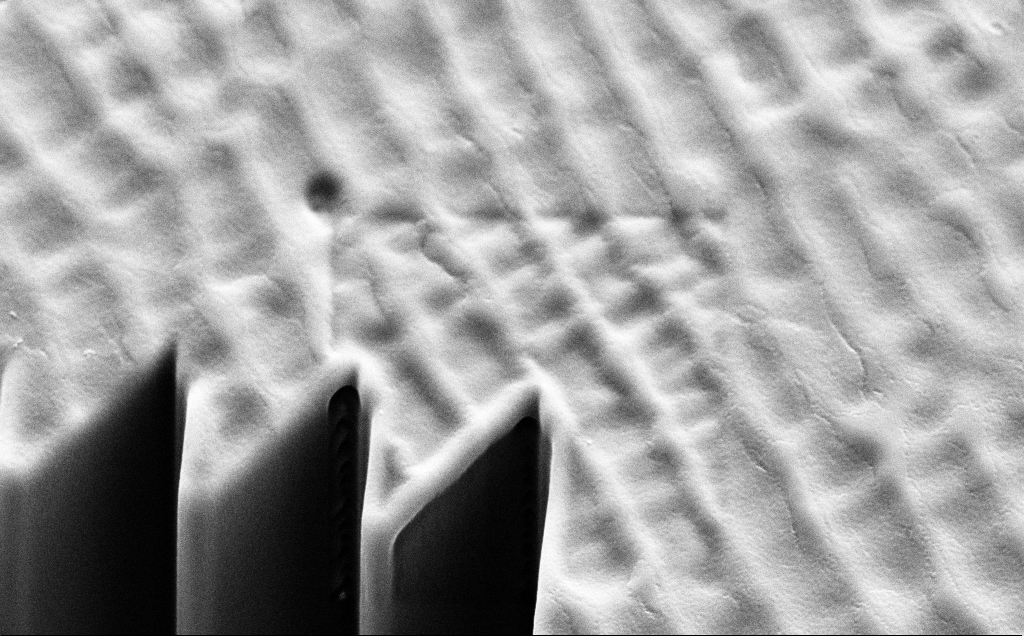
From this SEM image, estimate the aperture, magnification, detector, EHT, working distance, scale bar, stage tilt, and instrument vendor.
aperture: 30 µm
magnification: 5.84 K X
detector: SE2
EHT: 10 kV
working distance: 11 mm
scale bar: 10000 nm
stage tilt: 45°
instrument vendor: Zeiss